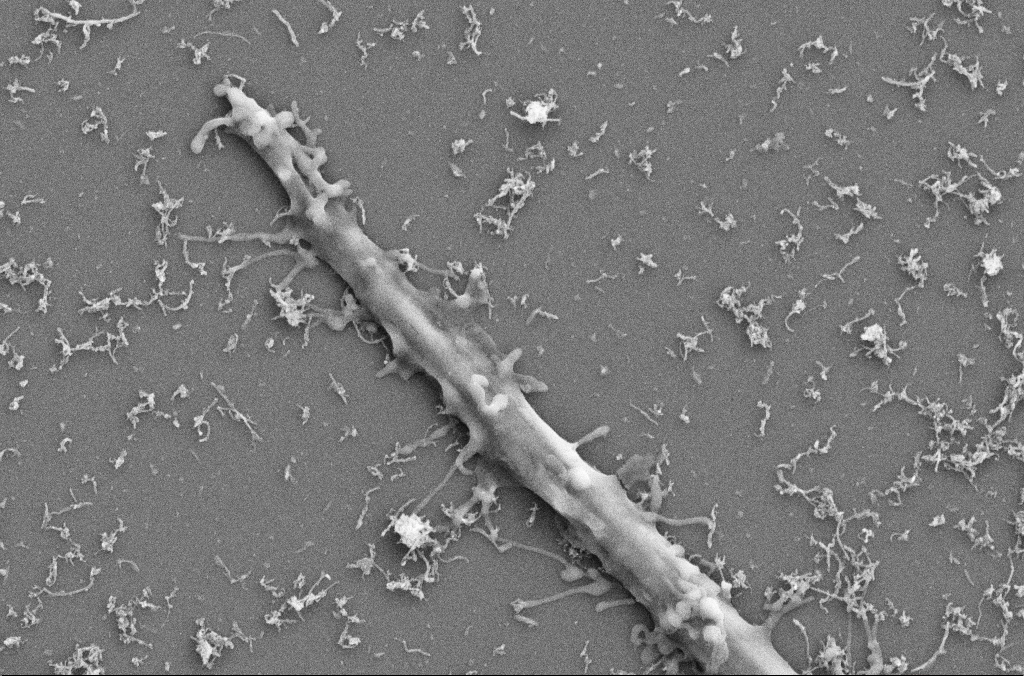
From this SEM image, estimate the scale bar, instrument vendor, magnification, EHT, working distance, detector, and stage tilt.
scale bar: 1000 nm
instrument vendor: Zeiss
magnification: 20 K X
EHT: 5 kV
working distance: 4 mm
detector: SE2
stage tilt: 0°